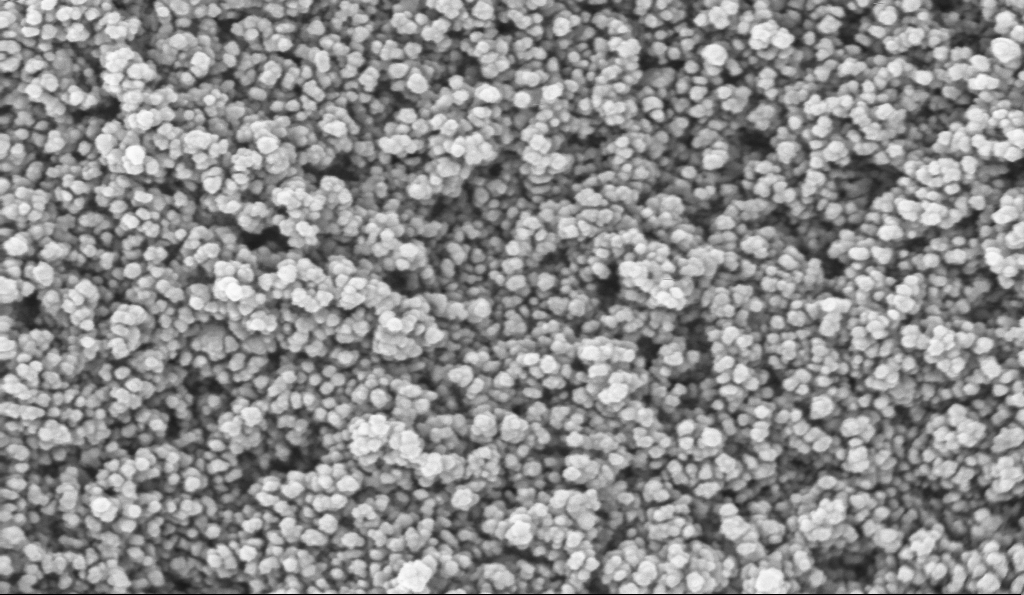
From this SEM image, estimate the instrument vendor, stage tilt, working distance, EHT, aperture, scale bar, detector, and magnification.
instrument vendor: Zeiss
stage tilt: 0°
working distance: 5.1 mm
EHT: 10 kV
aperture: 30 µm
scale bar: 100 nm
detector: InLens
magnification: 135 K X